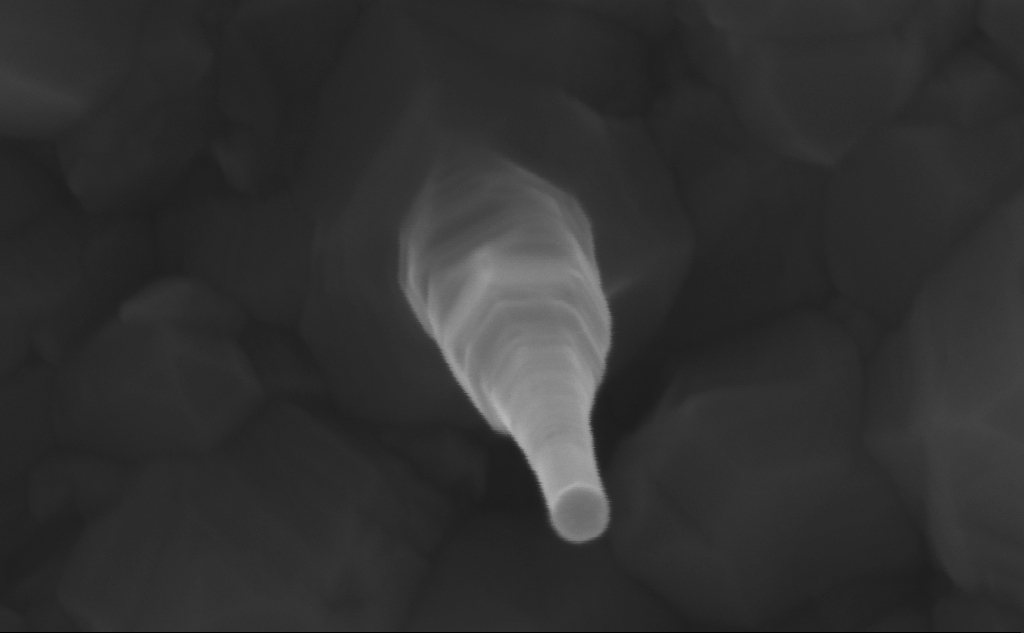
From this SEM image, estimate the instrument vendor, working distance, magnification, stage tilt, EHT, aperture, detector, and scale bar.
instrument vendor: Zeiss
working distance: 7 mm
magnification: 419.07 K X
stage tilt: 0°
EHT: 10 kV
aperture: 30 µm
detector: InLens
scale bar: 100 nm